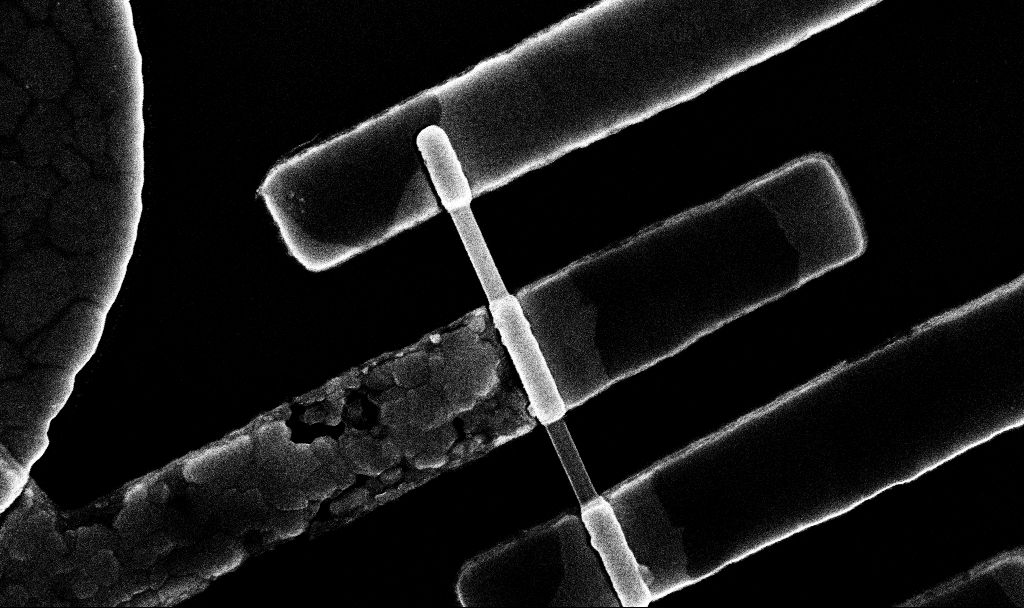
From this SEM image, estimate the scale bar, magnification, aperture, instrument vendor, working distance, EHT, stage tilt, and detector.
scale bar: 200 nm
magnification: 75.88 K X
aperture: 30 µm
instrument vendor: Zeiss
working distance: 6.8 mm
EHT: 10 kV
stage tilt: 0°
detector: InLens